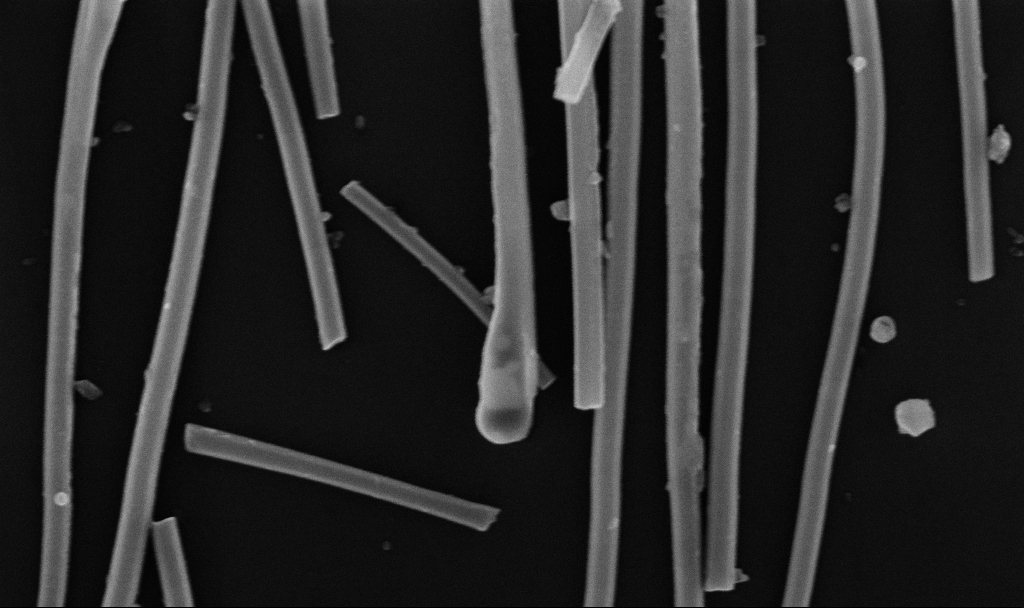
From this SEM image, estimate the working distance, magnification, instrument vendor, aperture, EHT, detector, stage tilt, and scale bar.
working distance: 6.7 mm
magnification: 100 K X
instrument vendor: Zeiss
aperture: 30 µm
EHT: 10 kV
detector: InLens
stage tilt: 0°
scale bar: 200 nm